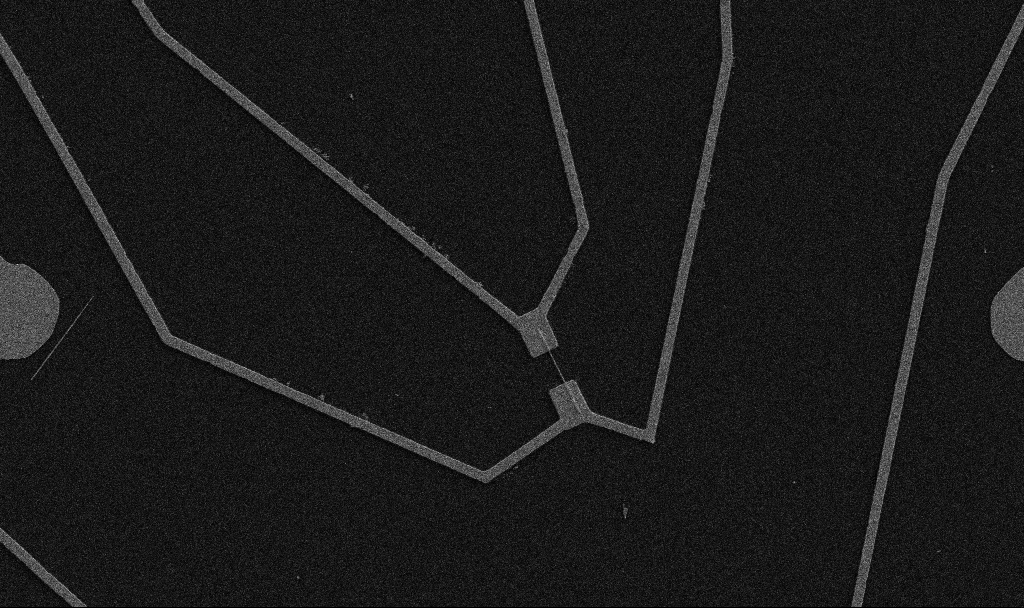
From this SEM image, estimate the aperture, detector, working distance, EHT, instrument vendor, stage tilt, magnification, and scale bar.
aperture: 30 µm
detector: SE2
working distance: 10.7 mm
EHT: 5 kV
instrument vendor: Zeiss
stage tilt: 0°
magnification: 5 K X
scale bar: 10000 nm